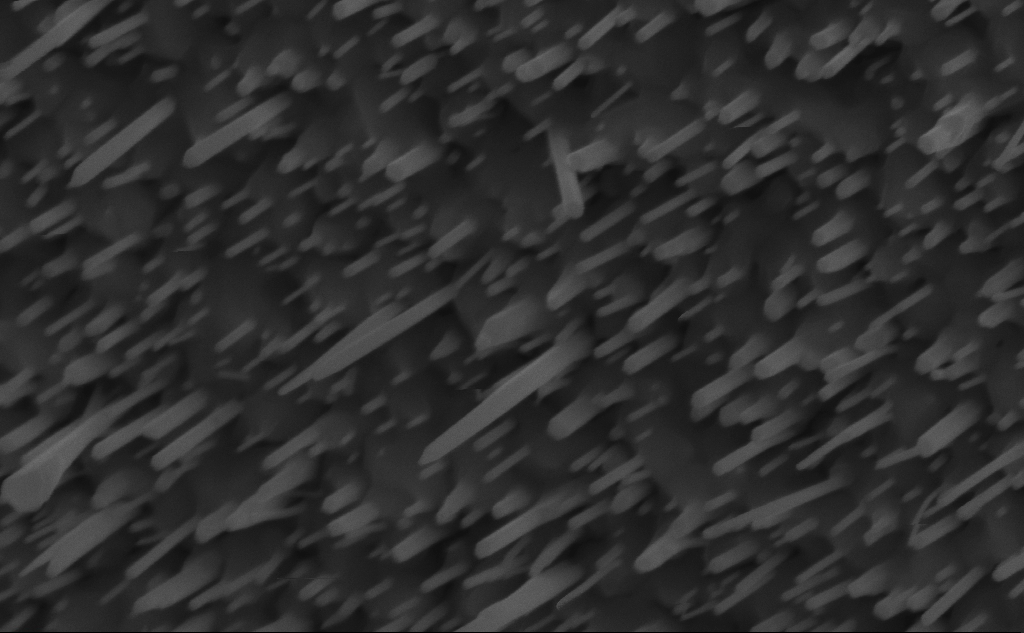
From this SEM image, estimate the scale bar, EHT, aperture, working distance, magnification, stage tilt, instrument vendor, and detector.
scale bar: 200 nm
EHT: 10 kV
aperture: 30 µm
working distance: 6 mm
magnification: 118.09 K X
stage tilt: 45°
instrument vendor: Zeiss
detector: InLens